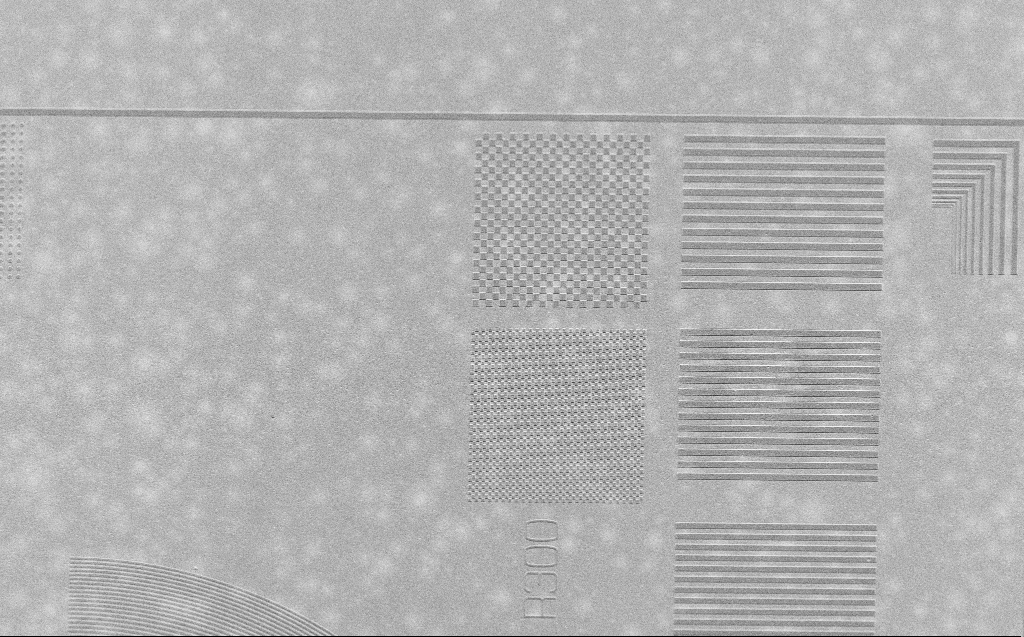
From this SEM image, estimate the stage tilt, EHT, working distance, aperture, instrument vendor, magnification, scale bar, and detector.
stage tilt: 30°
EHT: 2.5 kV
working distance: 4 mm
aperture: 30 µm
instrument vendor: Zeiss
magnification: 2.5 K X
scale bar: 20000 nm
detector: SE2